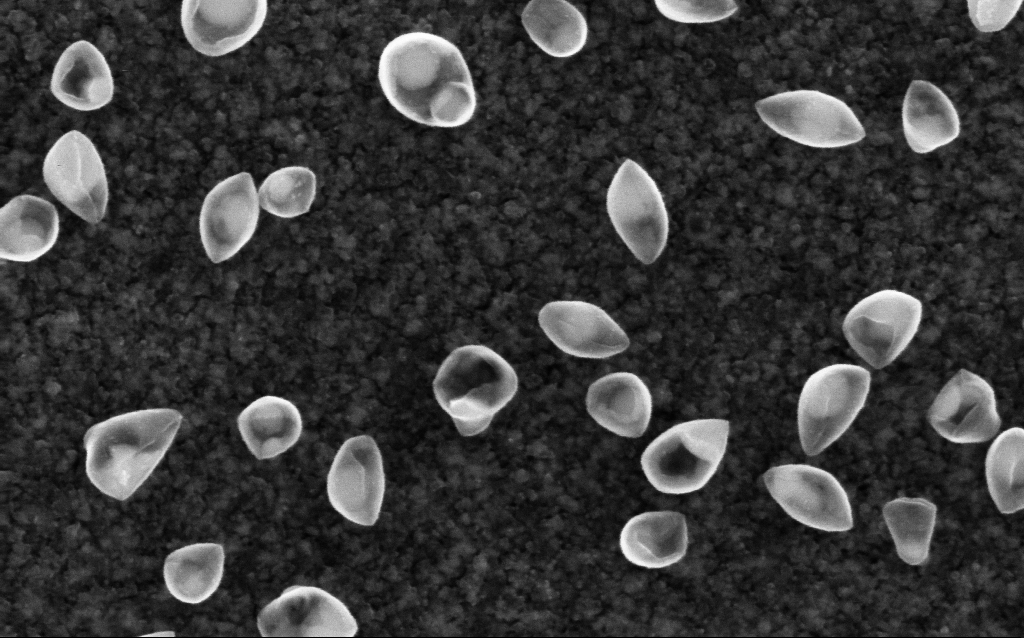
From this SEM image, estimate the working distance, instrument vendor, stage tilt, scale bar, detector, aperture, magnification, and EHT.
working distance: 2.6 mm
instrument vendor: Zeiss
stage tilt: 0°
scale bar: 100 nm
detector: InLens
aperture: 30 µm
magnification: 200 K X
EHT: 5 kV